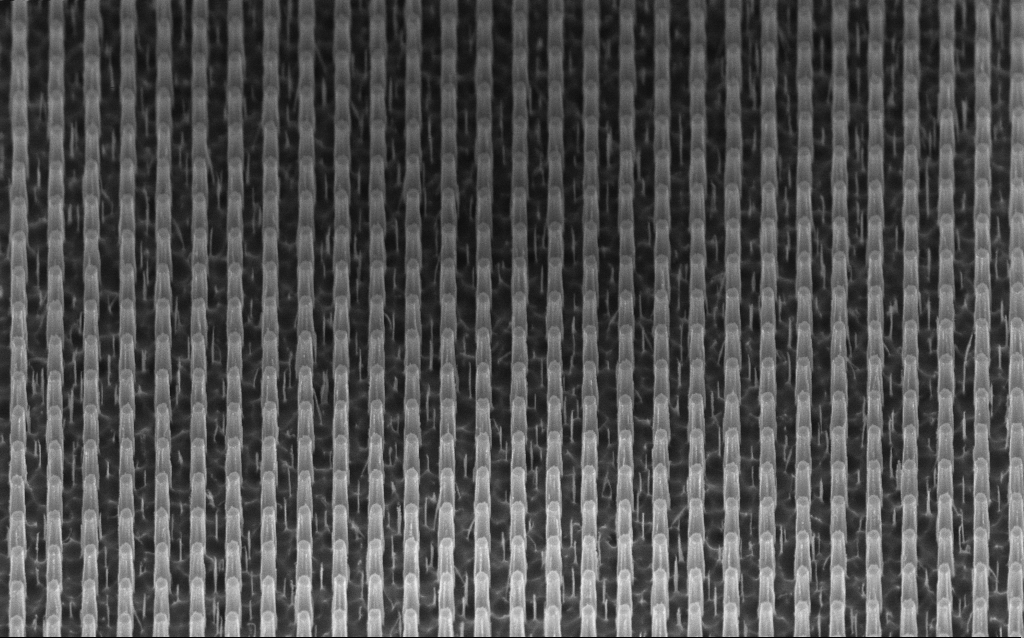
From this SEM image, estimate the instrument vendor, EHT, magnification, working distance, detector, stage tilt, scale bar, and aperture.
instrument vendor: Zeiss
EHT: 3 kV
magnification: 33.09 K X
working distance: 5 mm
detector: InLens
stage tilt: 45°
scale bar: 1000 nm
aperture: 30 µm